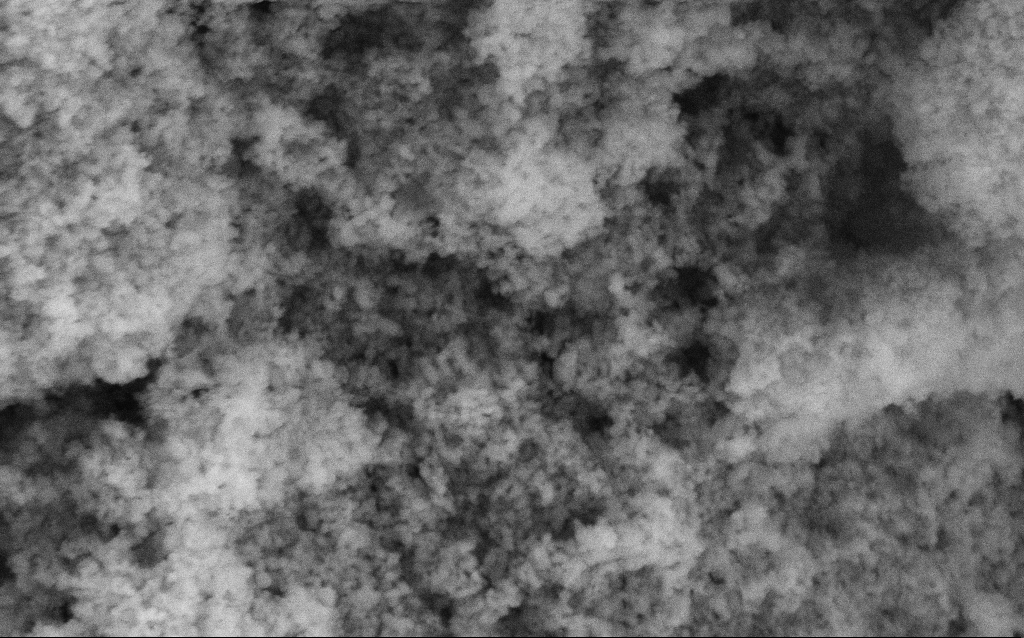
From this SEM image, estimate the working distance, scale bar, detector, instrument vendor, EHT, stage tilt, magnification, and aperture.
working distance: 4.6 mm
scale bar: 200 nm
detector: SE2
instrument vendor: Zeiss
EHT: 5 kV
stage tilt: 0°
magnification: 114.25 K X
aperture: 30 µm